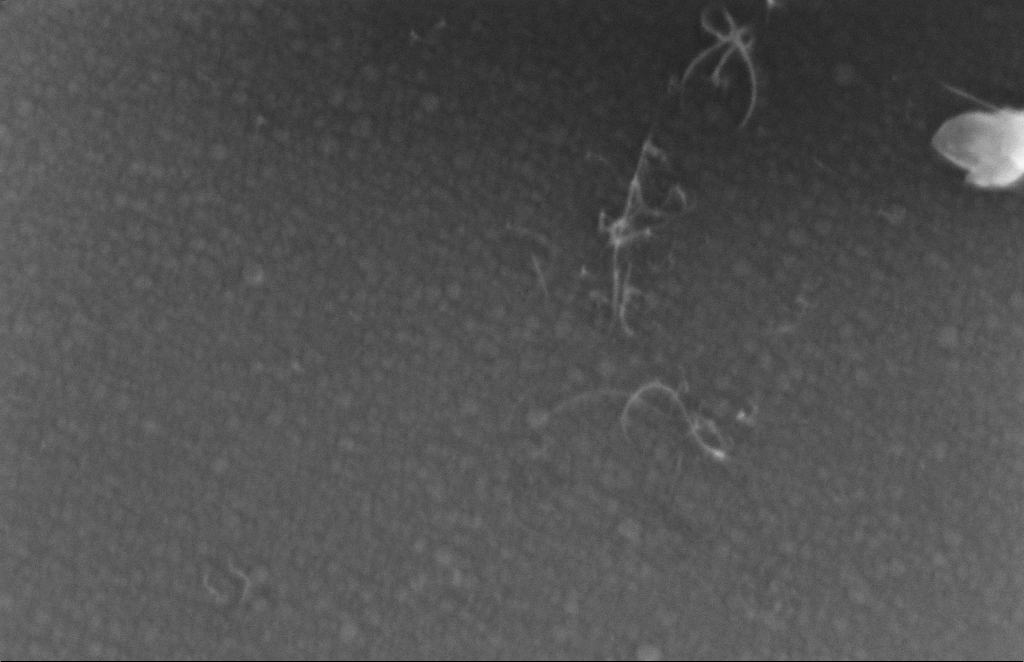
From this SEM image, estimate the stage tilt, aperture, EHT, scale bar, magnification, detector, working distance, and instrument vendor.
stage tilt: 0°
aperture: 30 µm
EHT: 5 kV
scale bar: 100 nm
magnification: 337.46 K X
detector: InLens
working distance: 5 mm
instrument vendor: Zeiss